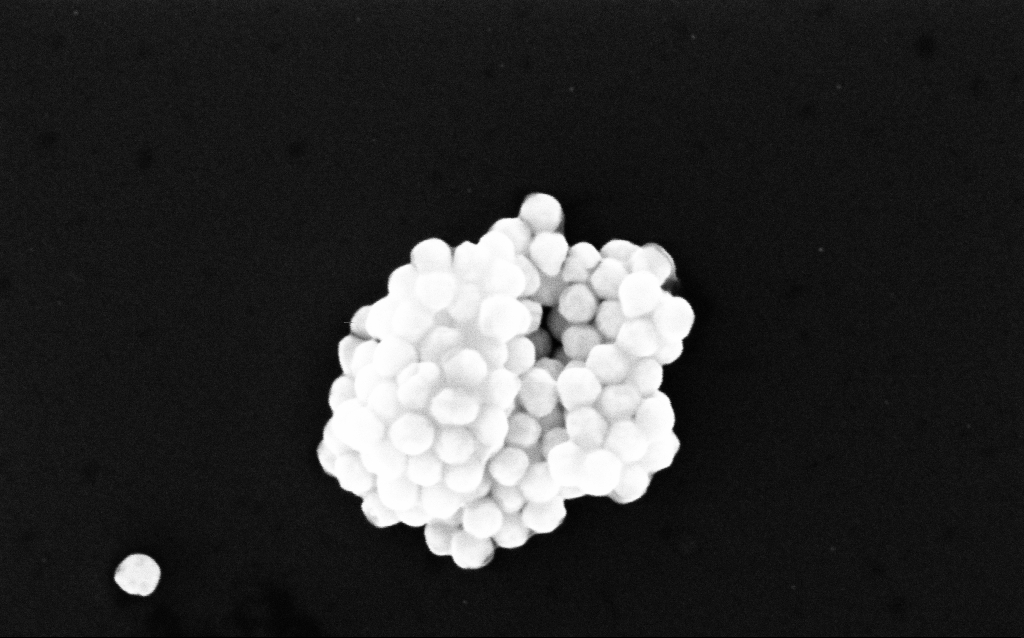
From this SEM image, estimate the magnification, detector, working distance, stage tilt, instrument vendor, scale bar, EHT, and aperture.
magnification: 249.7 K X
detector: InLens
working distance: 3.1 mm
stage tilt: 0°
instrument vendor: Zeiss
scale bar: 200 nm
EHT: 10 kV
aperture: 30 µm